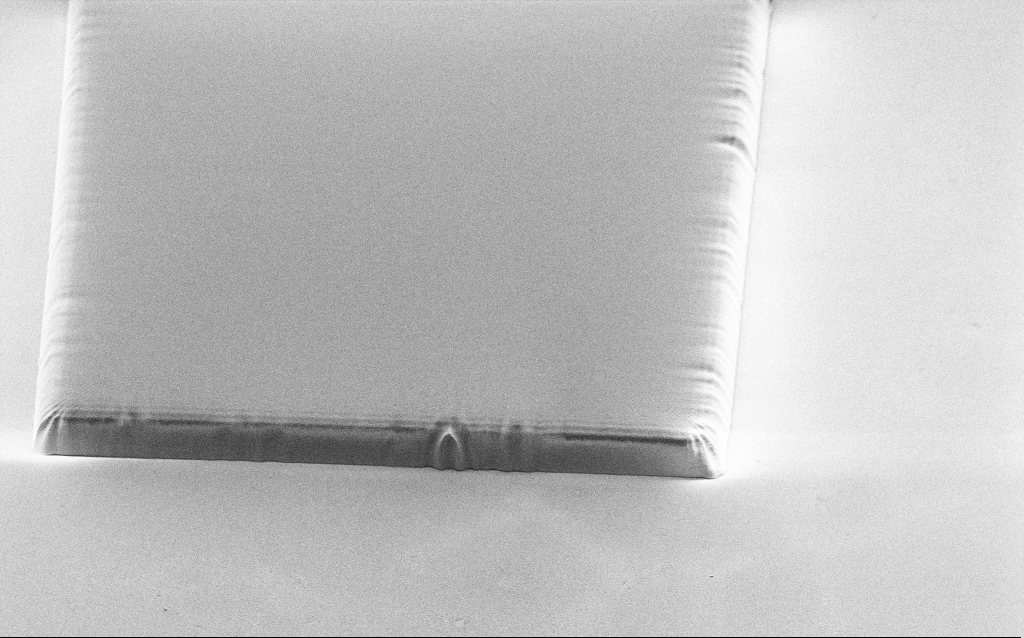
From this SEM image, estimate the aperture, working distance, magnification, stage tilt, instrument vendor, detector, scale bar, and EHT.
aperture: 30 µm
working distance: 8 mm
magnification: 2.94 K X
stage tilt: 45°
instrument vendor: Zeiss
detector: SE2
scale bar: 20000 nm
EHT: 1.2 kV